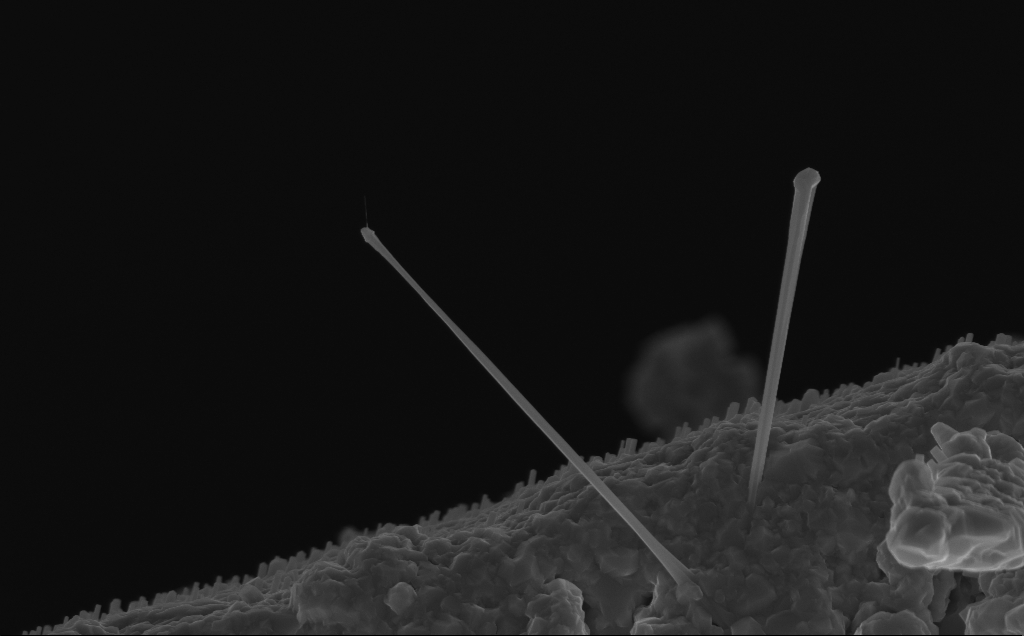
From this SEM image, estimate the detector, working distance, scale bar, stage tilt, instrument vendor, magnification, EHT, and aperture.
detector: InLens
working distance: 6 mm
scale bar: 1000 nm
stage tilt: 0°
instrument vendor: Zeiss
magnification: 21.22 K X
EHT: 10 kV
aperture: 30 µm